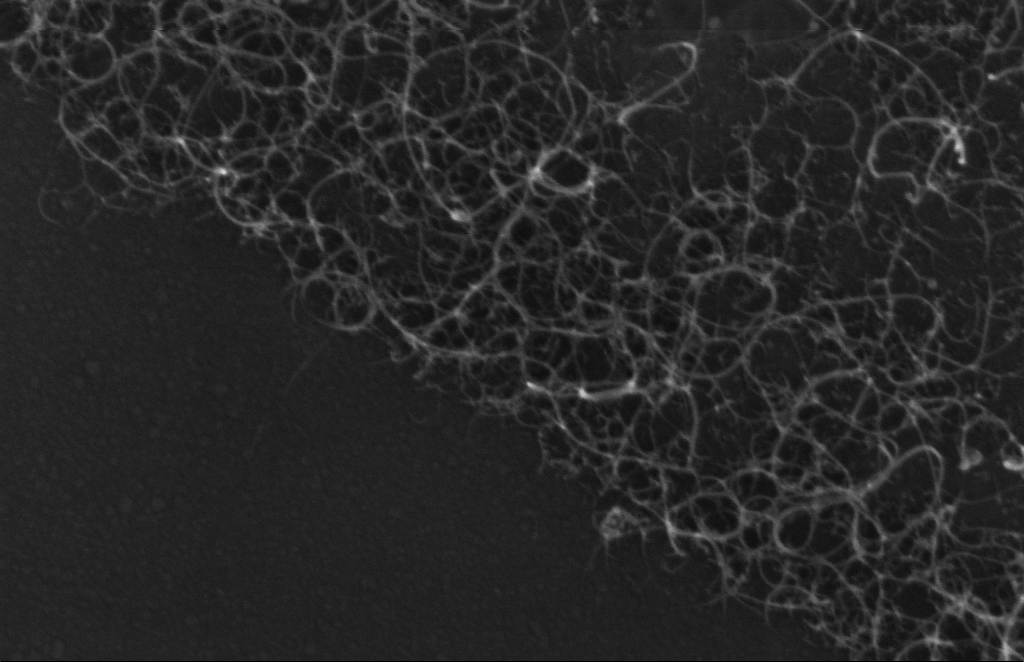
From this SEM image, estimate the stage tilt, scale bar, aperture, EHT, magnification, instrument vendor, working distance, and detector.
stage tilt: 0°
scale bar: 200 nm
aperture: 20 µm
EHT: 5 kV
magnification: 228.2 K X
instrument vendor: Zeiss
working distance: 5 mm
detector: InLens